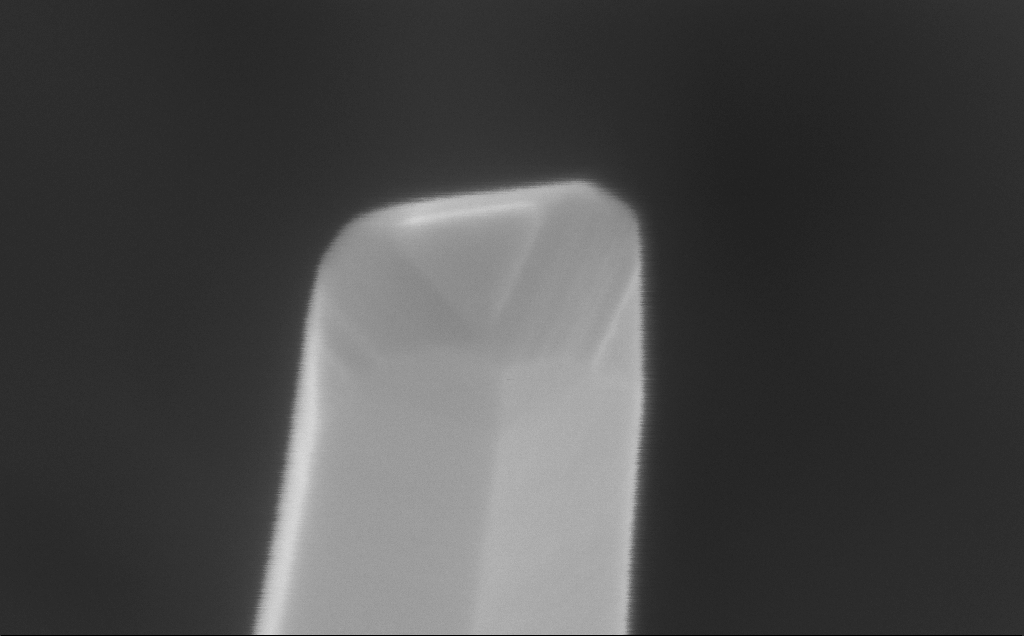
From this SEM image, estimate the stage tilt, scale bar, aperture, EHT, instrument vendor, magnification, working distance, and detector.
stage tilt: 0°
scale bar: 20 nm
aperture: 30 µm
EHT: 10 kV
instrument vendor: Zeiss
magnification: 735.17 K X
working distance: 5 mm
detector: InLens